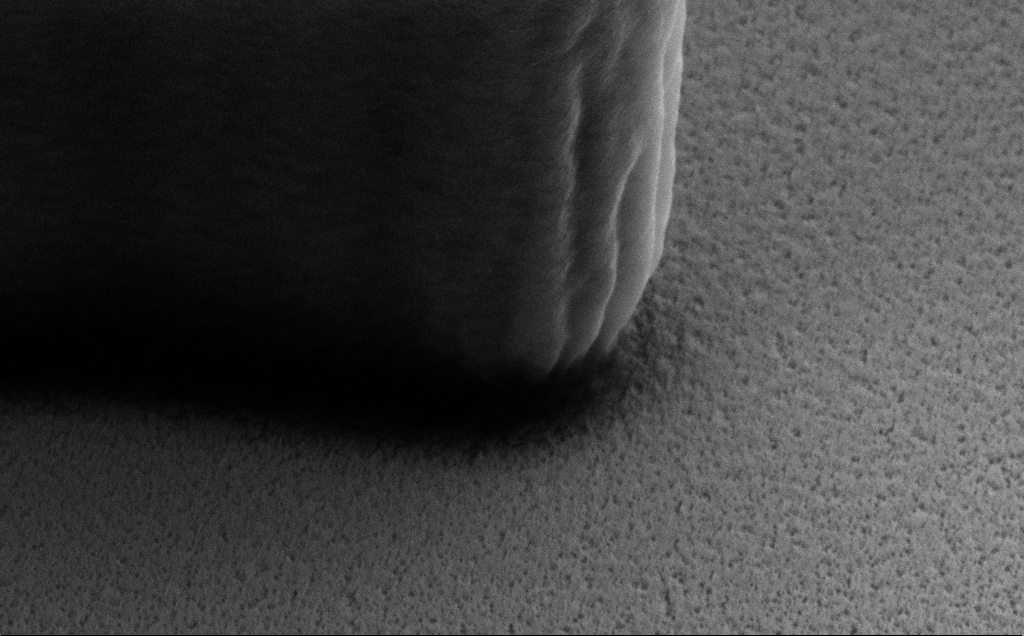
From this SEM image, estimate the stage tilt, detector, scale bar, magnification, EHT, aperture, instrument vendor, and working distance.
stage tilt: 40°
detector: SE2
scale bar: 200 nm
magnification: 74.49 K X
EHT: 5 kV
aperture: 30 µm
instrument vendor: Zeiss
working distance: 9 mm